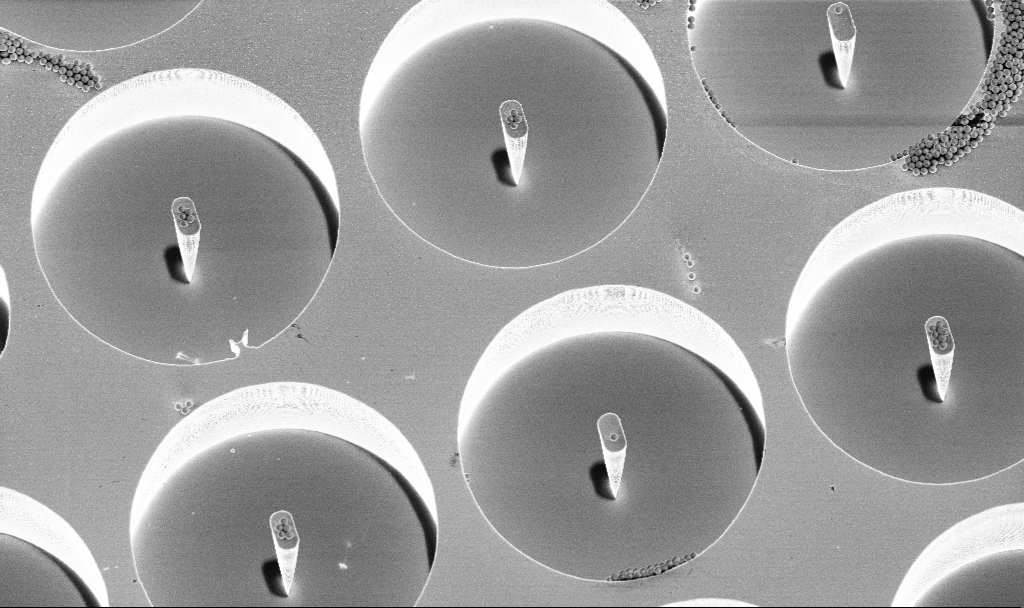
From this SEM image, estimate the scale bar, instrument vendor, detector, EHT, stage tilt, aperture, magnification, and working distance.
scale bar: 10000 nm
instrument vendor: Zeiss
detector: InLens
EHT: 4 kV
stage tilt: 15°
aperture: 30 µm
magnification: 3.81 K X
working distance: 4.7 mm